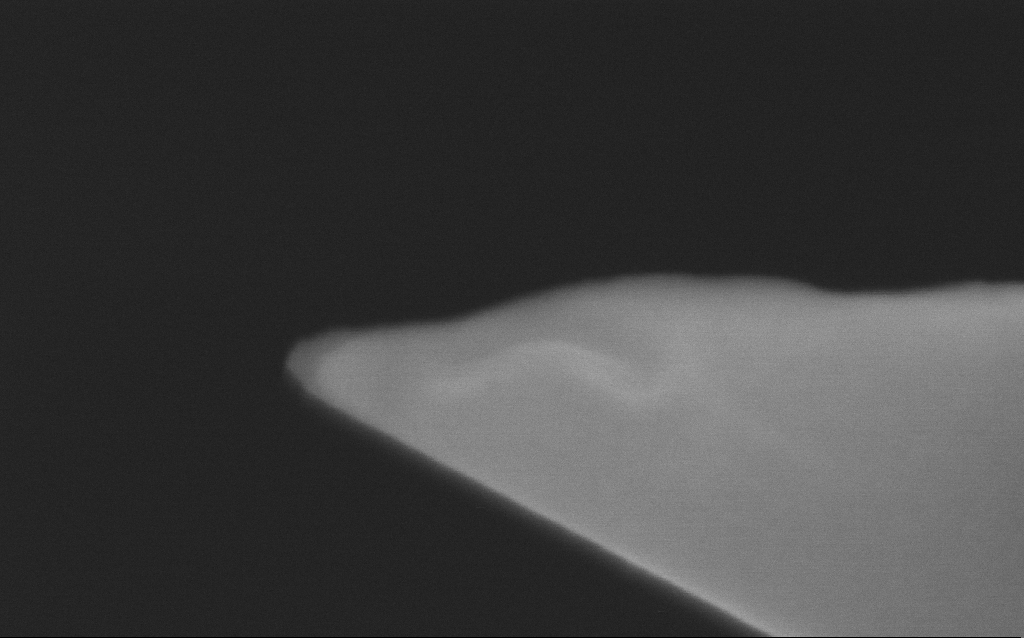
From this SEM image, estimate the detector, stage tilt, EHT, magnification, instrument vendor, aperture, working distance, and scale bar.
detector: InLens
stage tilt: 0°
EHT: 5 kV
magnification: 500 K X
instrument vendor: Zeiss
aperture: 30 µm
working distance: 4.7 mm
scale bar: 100 nm